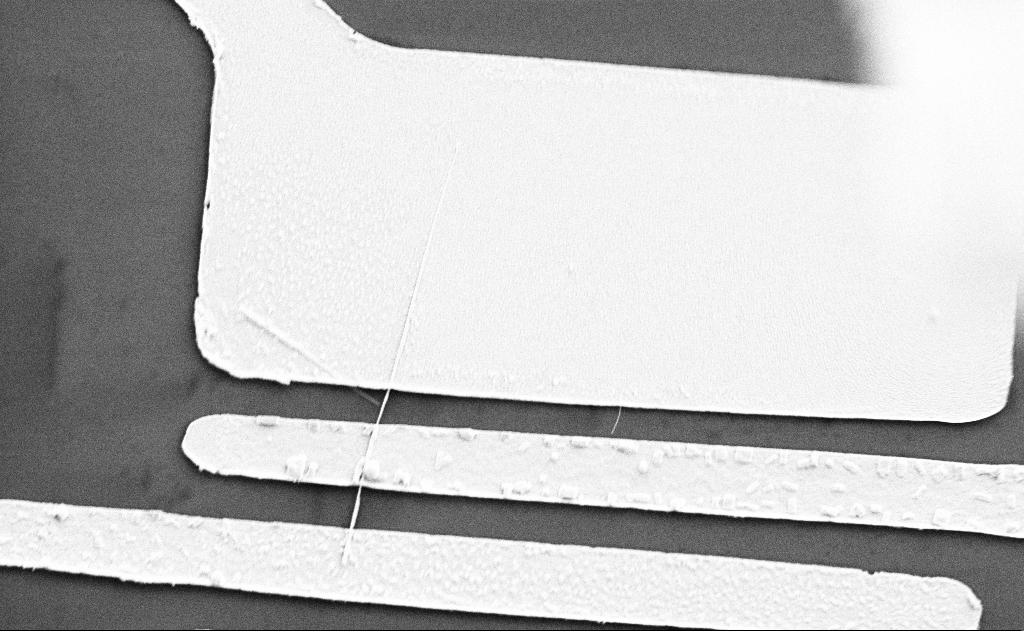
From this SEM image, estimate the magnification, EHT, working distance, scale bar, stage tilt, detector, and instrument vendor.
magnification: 10 K X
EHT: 5 kV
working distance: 15 mm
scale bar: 2000 nm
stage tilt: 0°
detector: SE2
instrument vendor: Zeiss